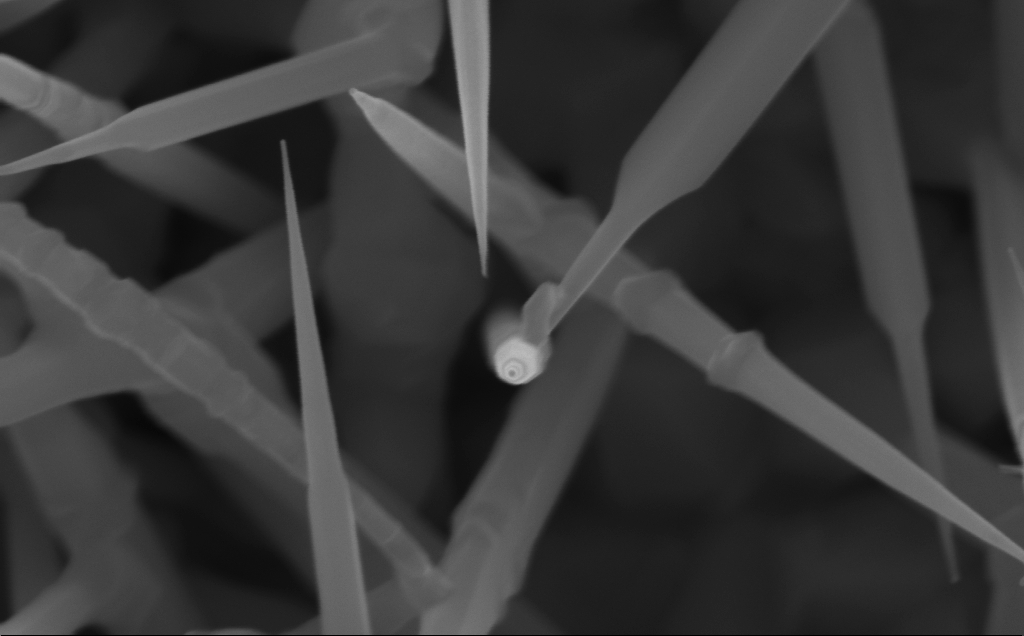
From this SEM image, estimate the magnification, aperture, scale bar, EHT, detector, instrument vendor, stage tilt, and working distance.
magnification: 150 K X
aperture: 30 µm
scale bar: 100 nm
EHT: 10 kV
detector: InLens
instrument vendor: Zeiss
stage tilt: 0°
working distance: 4 mm